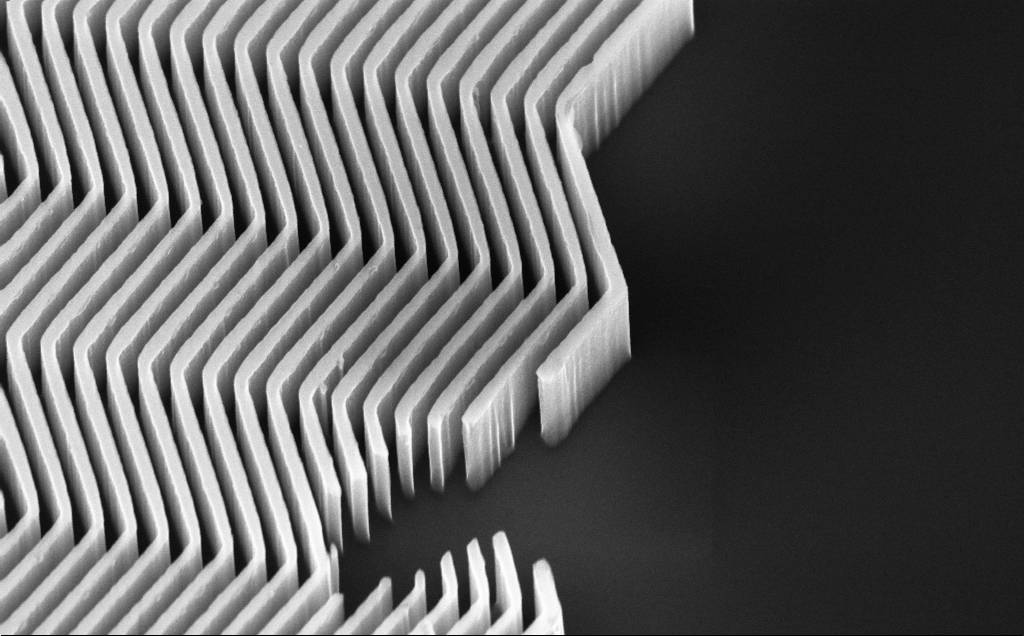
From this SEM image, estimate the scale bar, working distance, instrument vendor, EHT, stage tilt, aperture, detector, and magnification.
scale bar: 1000 nm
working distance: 6 mm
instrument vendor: Zeiss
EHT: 10 kV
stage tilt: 45°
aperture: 30 µm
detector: InLens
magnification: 60.5 K X